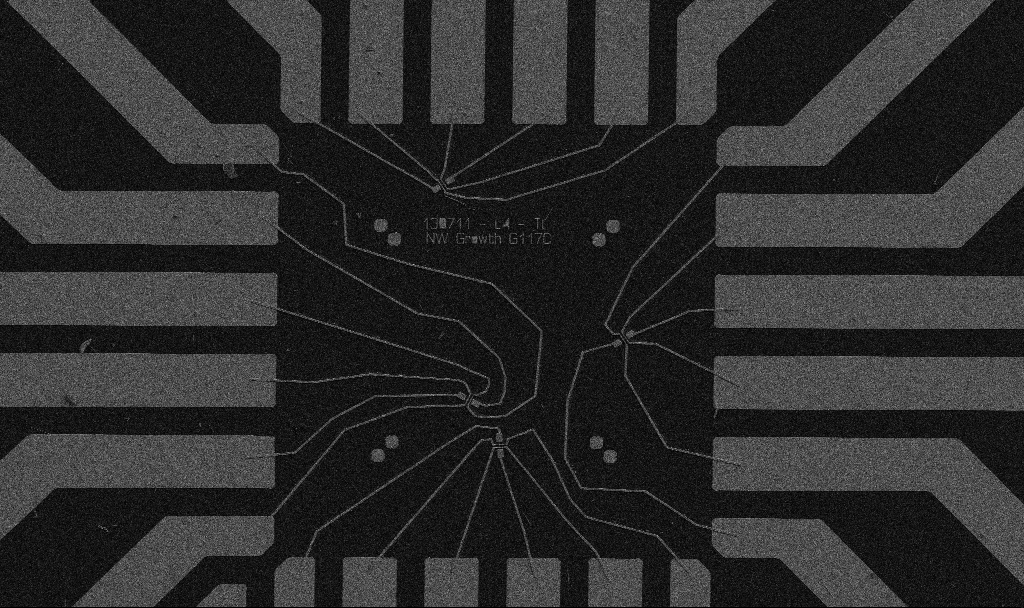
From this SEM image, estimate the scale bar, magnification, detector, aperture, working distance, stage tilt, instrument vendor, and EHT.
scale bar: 20000 nm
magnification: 1 K X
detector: SE2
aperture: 30 µm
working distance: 10.7 mm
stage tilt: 0°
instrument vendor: Zeiss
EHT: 5 kV